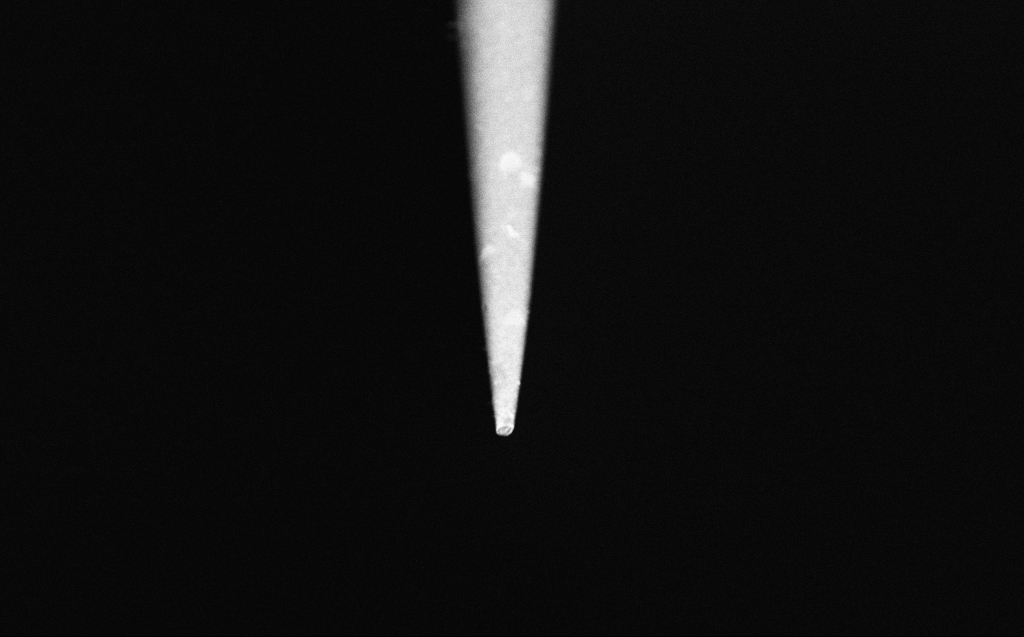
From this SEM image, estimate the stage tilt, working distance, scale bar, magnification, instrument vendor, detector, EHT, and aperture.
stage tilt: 45°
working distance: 4 mm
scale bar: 2000 nm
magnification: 10 K X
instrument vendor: Zeiss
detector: InLens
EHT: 4 kV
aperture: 30 µm